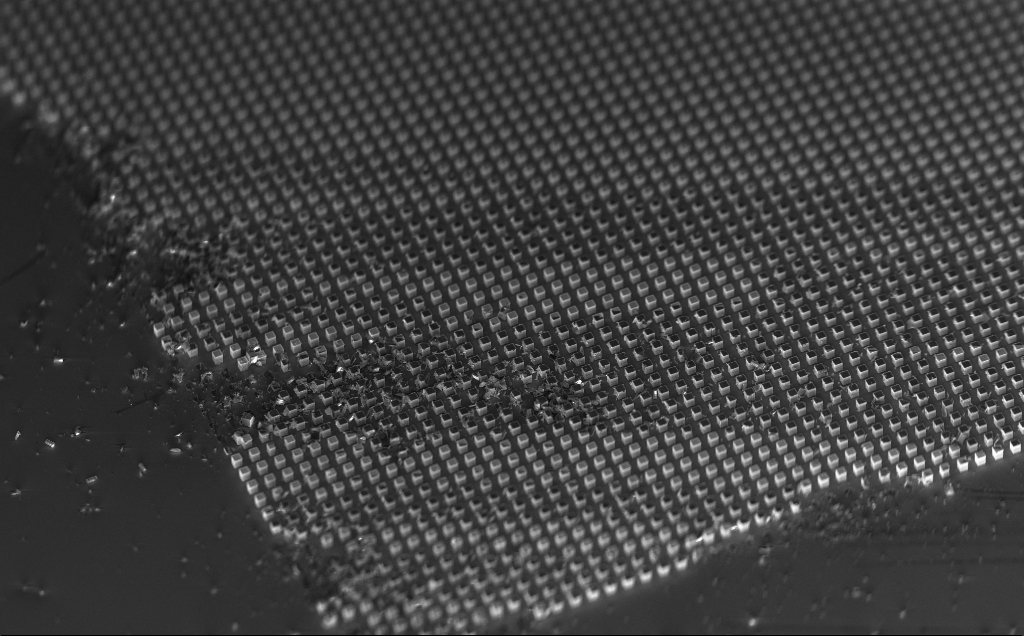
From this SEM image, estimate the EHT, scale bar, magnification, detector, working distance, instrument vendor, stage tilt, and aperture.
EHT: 10 kV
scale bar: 100000 nm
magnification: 0.354 K X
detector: InLens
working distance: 11 mm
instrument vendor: Zeiss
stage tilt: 48.7°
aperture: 120 µm